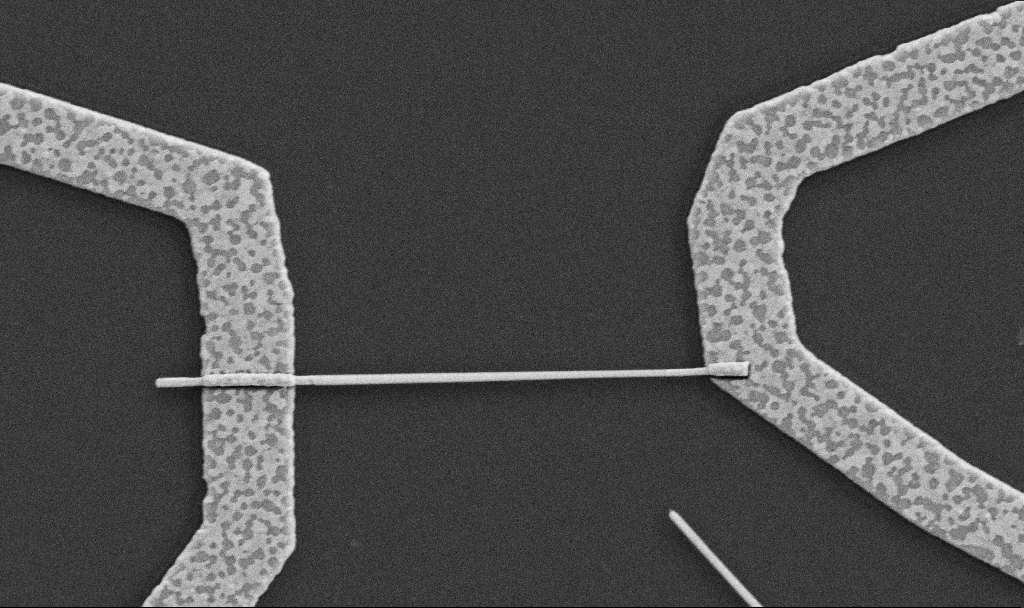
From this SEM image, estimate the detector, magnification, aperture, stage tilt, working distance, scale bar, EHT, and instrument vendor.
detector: SE2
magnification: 30 K X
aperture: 30 µm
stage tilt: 0°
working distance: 8.7 mm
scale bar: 1000 nm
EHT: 5 kV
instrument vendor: Zeiss